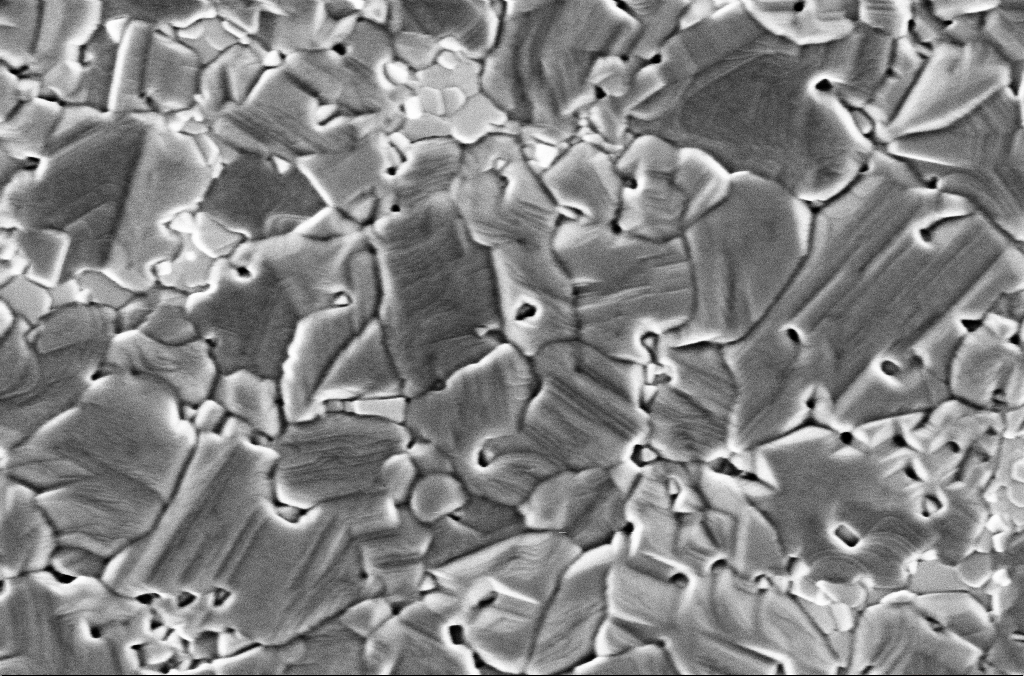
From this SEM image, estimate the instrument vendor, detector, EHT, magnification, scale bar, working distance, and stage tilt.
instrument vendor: Zeiss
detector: InLens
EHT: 2 kV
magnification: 70 K X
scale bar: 200 nm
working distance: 3 mm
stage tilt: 0°